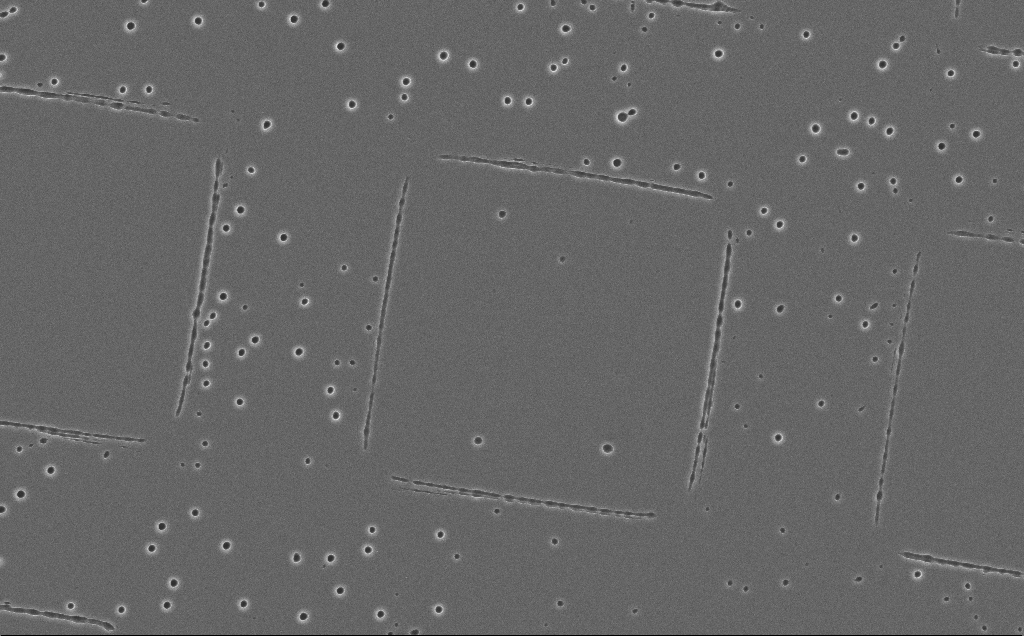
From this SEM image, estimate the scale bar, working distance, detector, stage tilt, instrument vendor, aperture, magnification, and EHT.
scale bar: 20000 nm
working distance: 16 mm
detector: SE2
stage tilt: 0°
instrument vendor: Zeiss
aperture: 30 µm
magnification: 1.34 K X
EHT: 10 kV